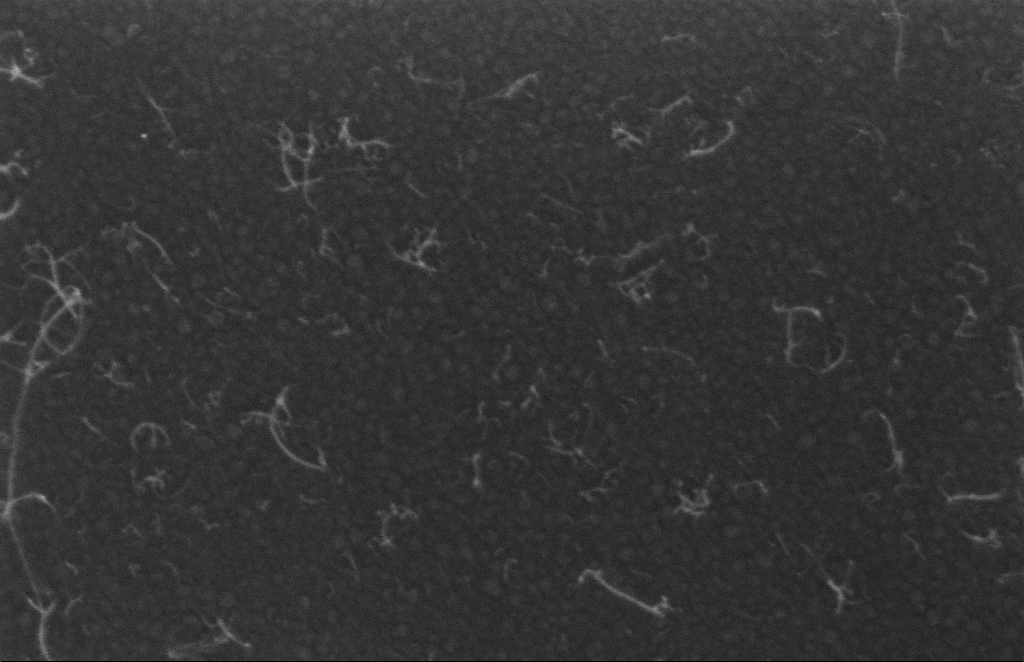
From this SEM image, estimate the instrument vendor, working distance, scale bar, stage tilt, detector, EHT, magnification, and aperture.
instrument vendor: Zeiss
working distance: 8 mm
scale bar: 100 nm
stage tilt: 0°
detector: InLens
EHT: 5 kV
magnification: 270.4 K X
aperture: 20 µm